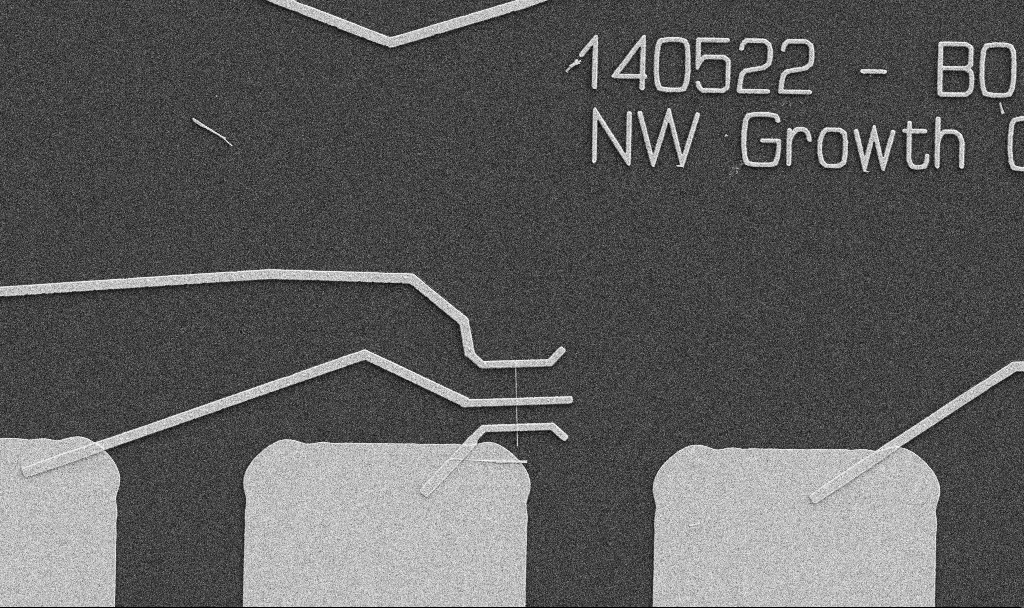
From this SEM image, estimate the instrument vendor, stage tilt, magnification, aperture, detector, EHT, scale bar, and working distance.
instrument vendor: Zeiss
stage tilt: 0°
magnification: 5 K X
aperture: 30 µm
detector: SE2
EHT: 5 kV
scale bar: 10000 nm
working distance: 10.7 mm